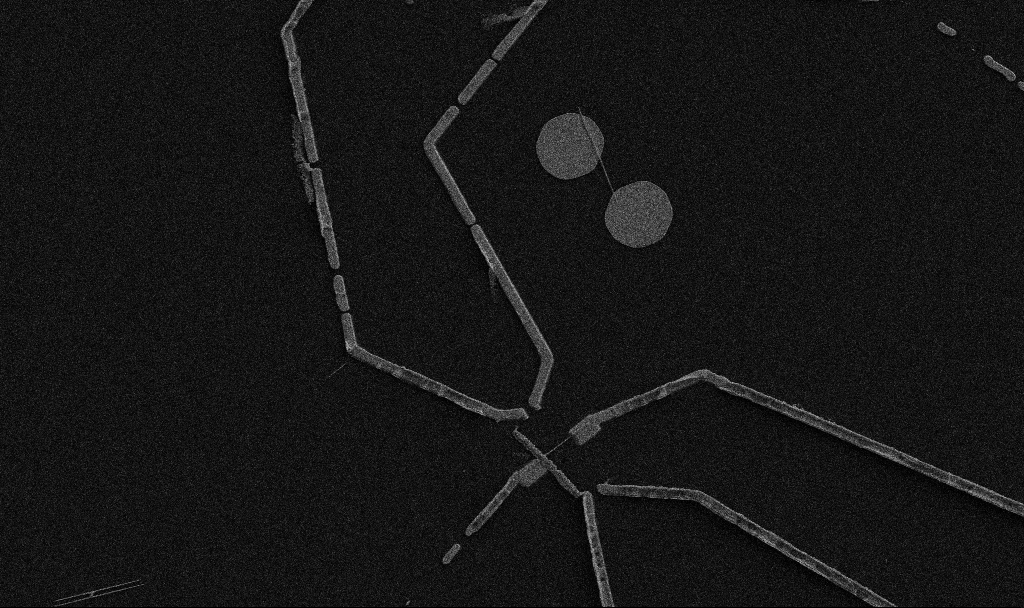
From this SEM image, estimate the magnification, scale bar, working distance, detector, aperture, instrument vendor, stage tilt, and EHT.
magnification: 5 K X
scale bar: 10000 nm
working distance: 10.7 mm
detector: SE2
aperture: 30 µm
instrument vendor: Zeiss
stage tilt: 0°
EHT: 5 kV